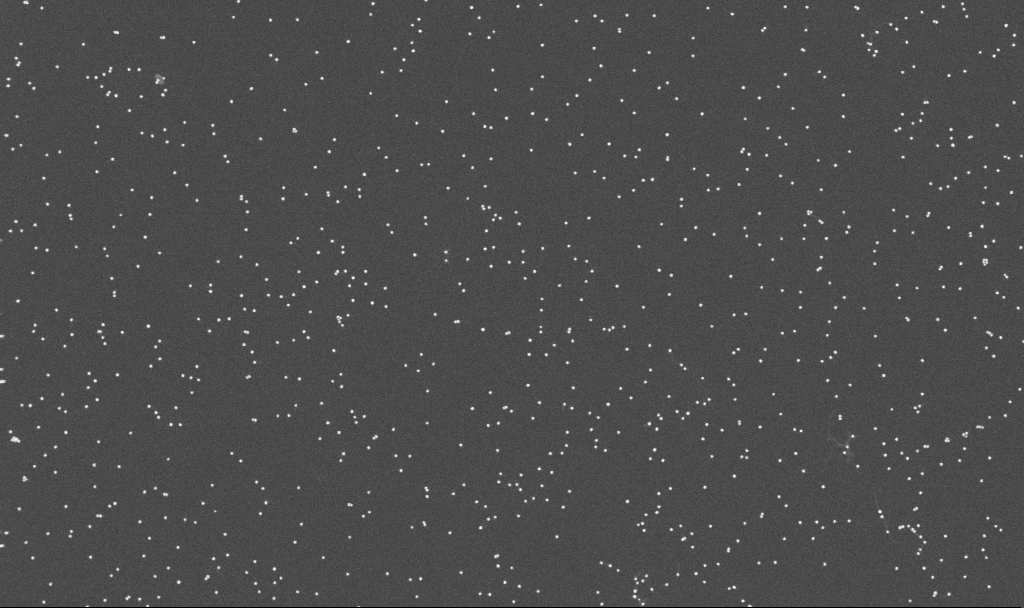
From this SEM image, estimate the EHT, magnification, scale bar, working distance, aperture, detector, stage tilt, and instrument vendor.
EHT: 10 kV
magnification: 100 K X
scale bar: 200 nm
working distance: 3.3 mm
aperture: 30 µm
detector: InLens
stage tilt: -0°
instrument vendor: Zeiss